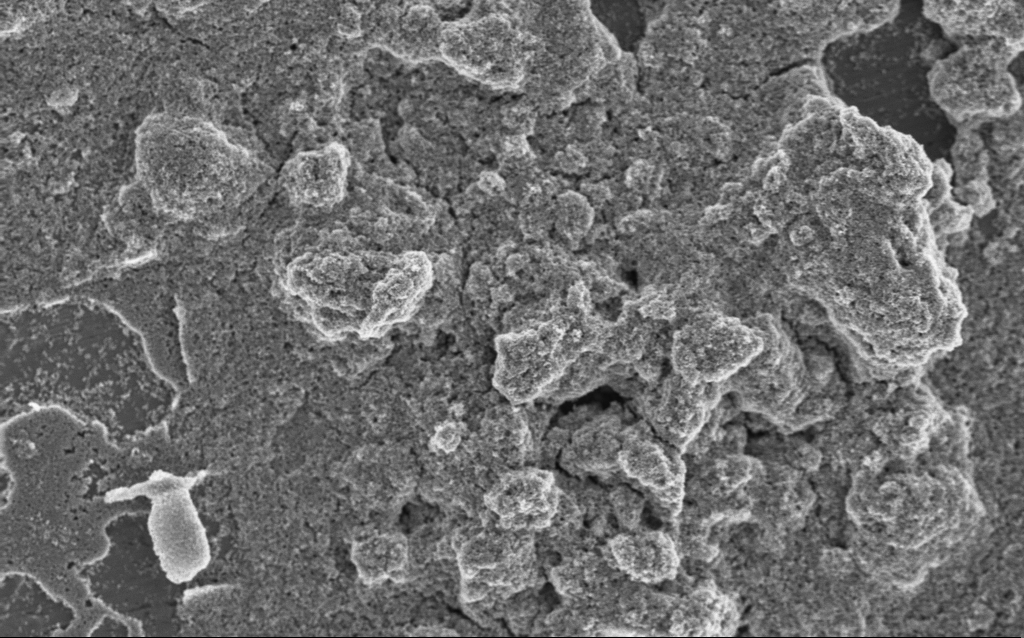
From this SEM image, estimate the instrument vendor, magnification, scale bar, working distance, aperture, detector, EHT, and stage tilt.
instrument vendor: Zeiss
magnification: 6.41 K X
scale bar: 10000 nm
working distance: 4.2 mm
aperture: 30 µm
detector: InLens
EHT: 5 kV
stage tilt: -0°